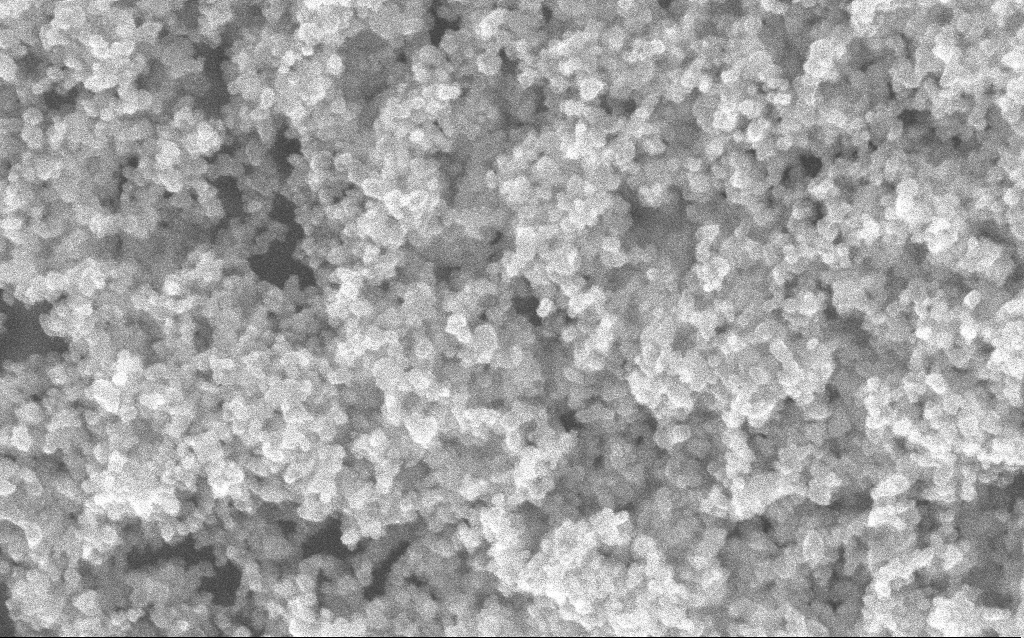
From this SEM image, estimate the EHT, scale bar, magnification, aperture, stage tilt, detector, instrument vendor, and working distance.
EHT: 10 kV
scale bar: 100 nm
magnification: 204.13 K X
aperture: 30 µm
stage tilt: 0°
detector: InLens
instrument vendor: Zeiss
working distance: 2.5 mm